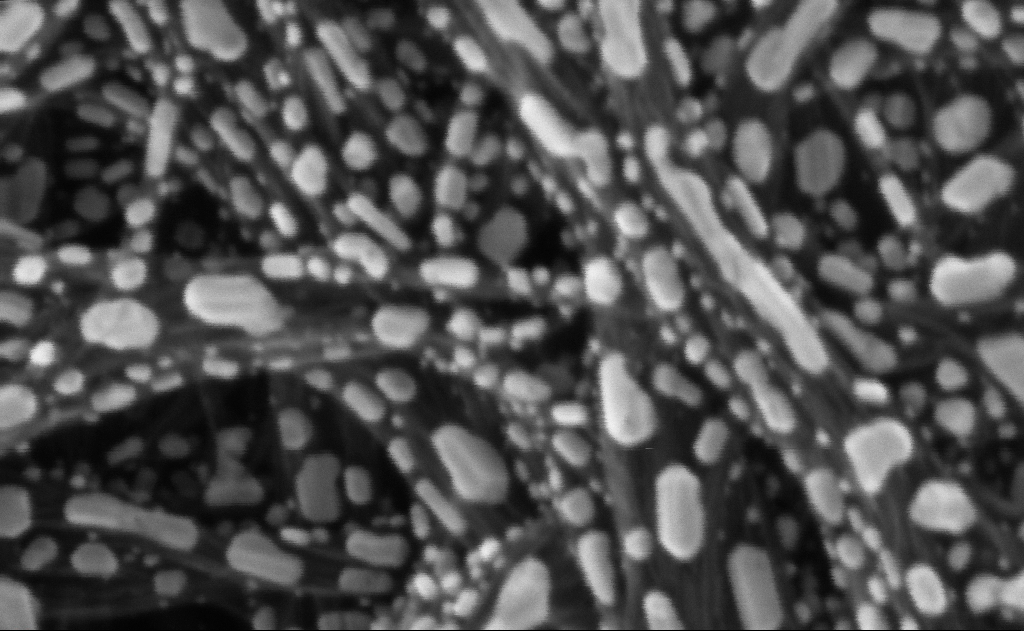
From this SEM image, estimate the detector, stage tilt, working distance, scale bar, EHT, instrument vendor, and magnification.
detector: InLens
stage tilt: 0°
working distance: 3 mm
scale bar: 20 nm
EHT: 10 kV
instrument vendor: Zeiss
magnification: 834.07 K X